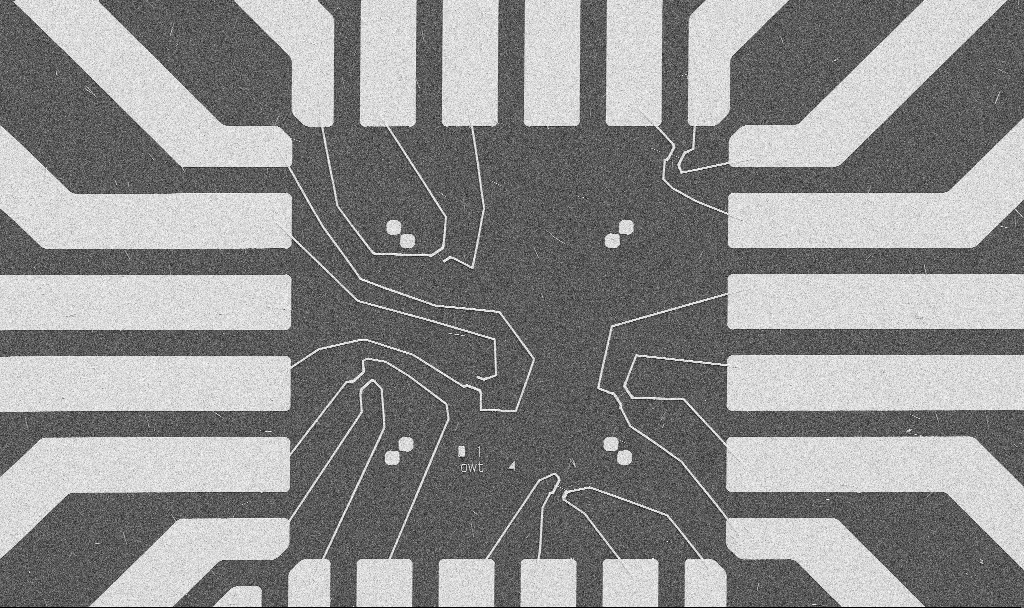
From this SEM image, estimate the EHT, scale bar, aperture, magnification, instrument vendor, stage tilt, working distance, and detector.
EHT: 5 kV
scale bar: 20000 nm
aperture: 30 µm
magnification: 1 K X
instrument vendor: Zeiss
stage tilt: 0°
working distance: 10.7 mm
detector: SE2